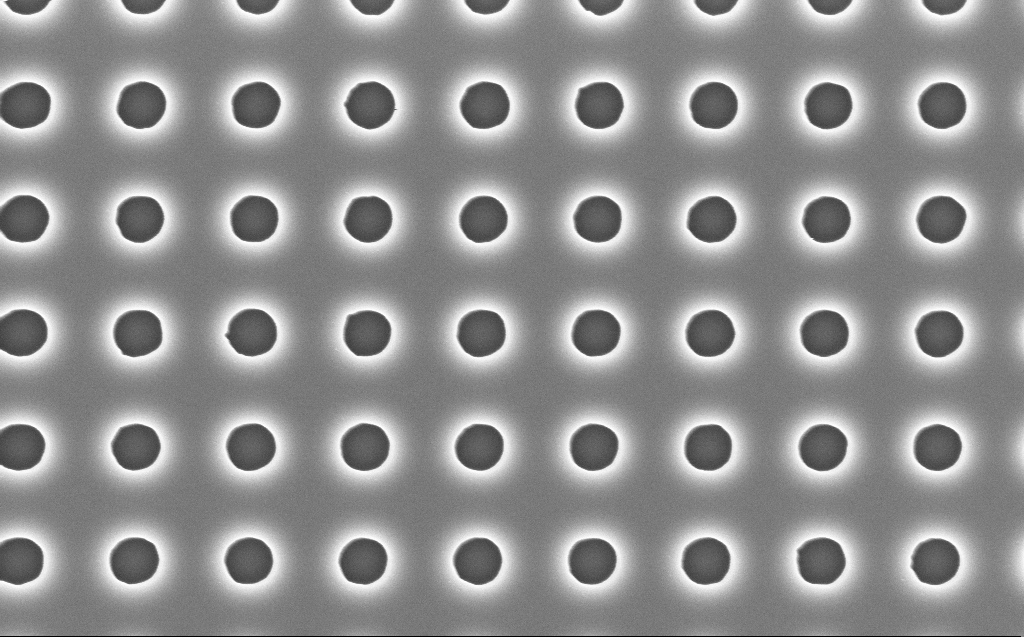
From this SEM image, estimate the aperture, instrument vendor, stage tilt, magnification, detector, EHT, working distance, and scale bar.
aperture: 30 µm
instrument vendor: Zeiss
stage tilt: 0°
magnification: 40 K X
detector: InLens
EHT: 5 kV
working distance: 7 mm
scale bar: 1000 nm